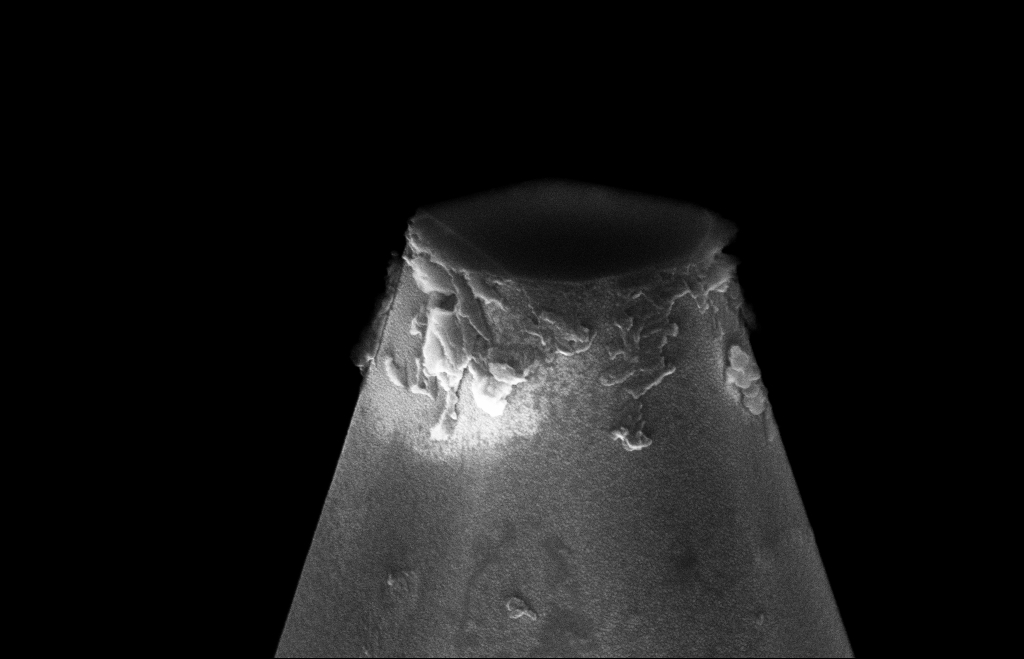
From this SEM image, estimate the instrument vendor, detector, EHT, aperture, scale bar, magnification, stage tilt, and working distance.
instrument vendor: Zeiss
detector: InLens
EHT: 10 kV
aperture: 30 µm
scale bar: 100 nm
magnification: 80.48 K X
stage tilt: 70°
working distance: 8 mm